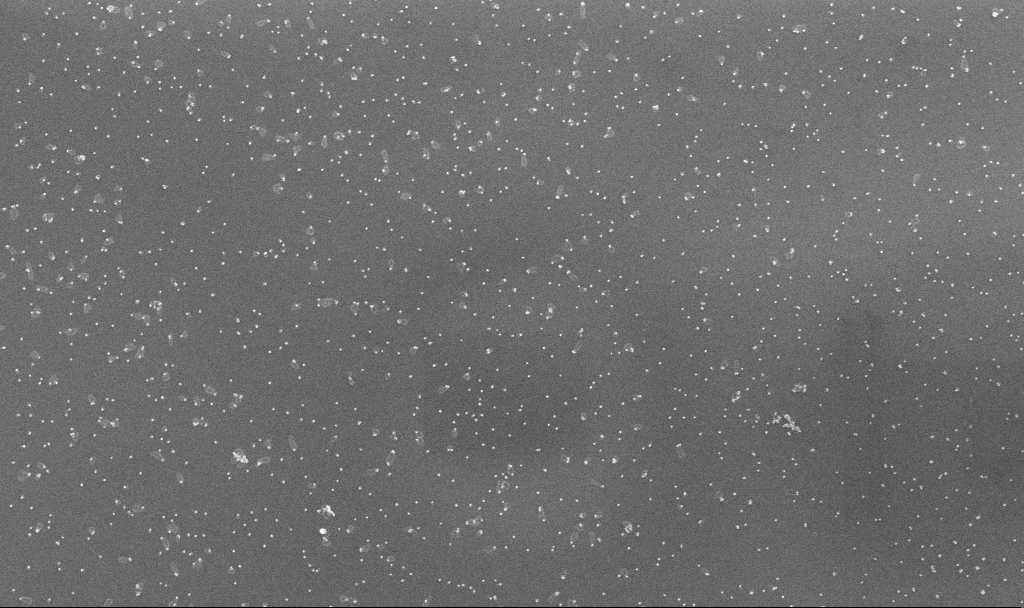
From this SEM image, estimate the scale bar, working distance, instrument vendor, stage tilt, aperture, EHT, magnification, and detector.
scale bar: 1000 nm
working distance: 3.3 mm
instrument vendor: Zeiss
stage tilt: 0°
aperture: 30 µm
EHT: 10 kV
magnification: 70 K X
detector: InLens